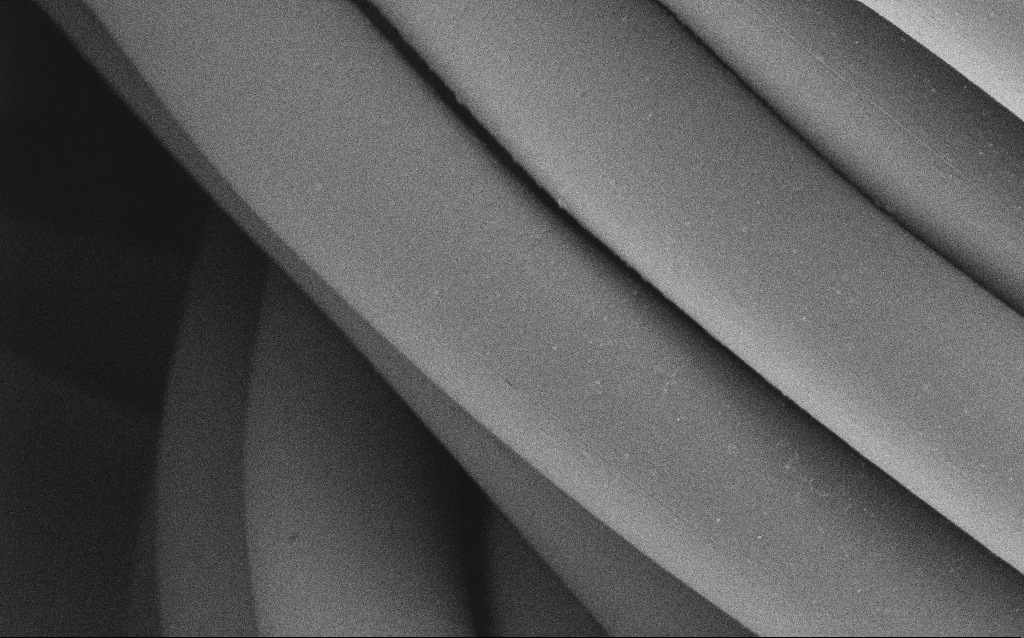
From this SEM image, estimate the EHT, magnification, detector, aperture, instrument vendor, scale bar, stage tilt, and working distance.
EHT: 1 kV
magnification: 3.31 K X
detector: SE2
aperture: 30 µm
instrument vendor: Zeiss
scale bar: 20000 nm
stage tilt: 0°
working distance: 4 mm